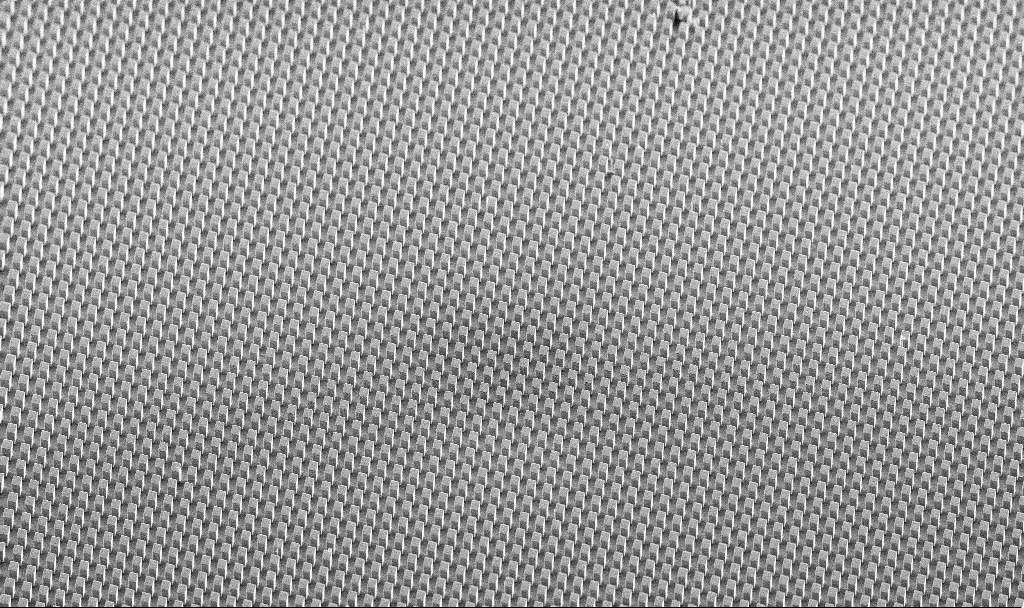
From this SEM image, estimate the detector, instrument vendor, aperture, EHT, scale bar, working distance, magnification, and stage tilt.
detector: SE2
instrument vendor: Zeiss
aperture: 30 µm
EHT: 5 kV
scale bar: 100000 nm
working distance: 10.1 mm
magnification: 0.383 K X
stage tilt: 45°